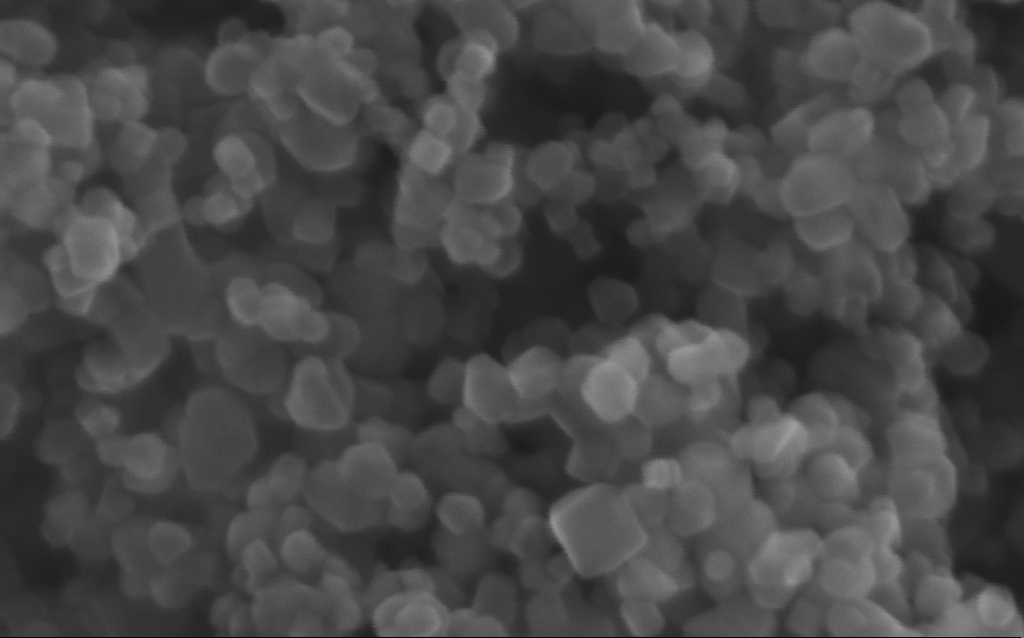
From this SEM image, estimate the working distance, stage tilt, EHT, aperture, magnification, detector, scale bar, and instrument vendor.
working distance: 2.7 mm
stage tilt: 0°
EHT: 10 kV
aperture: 30 µm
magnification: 716 K X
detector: InLens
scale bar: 100 nm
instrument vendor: Zeiss